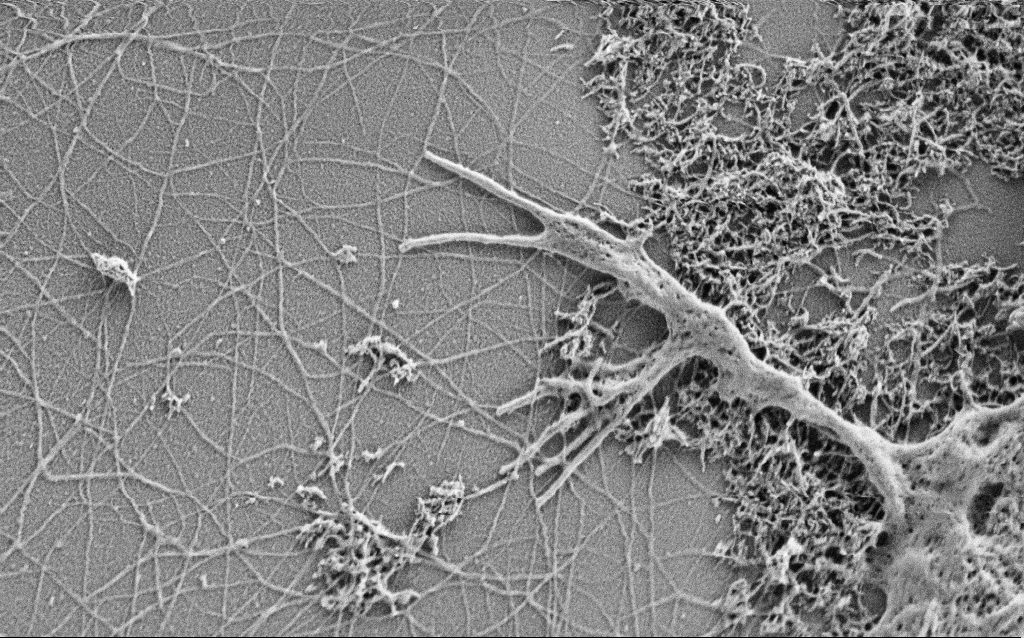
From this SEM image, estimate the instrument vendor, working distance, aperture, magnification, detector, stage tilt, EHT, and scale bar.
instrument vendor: Zeiss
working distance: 4 mm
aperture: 30 µm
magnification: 25 K X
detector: SE2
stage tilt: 0°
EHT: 1 kV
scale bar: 2000 nm